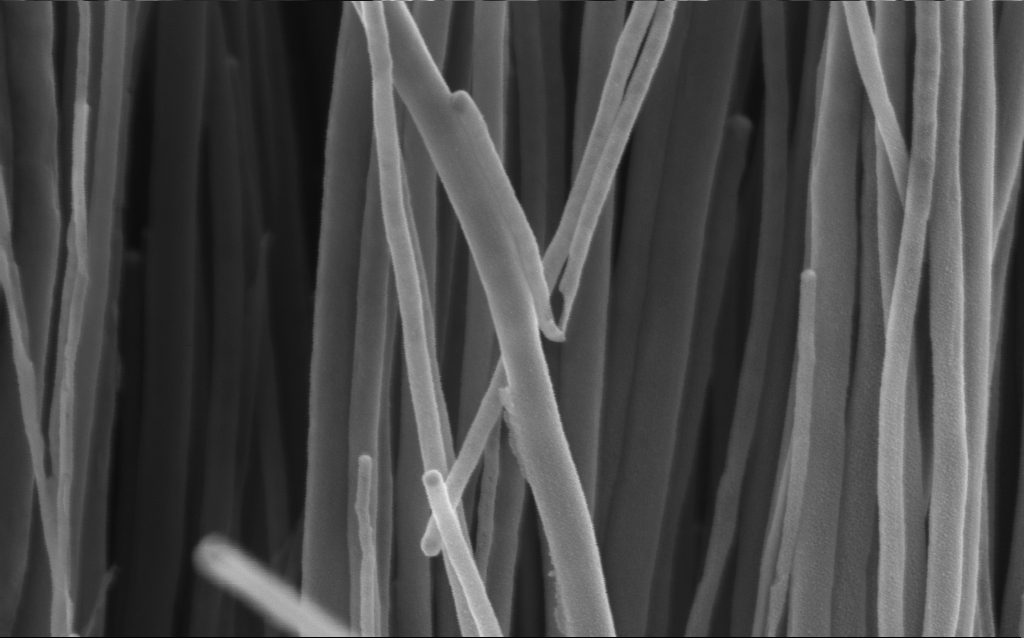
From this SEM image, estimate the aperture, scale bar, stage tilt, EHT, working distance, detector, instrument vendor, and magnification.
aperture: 30 µm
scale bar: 200 nm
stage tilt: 0°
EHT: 3 kV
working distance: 3 mm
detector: InLens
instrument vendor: Zeiss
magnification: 106.23 K X